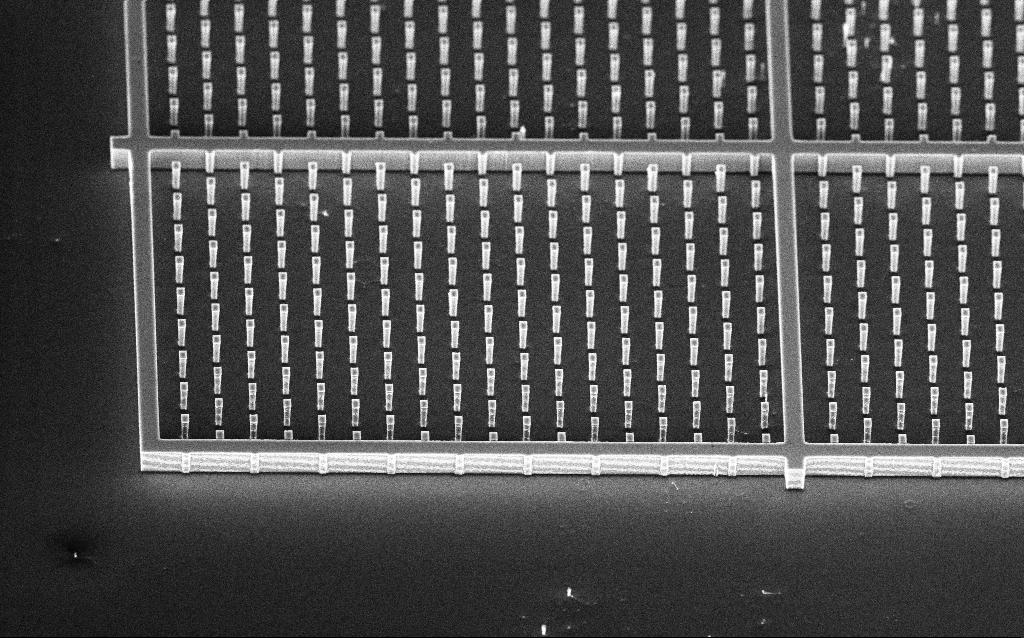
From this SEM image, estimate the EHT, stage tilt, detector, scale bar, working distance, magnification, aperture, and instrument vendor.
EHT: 10 kV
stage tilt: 45°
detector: InLens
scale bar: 10000 nm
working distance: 4.8 mm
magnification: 1.39 K X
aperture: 30 µm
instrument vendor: Zeiss